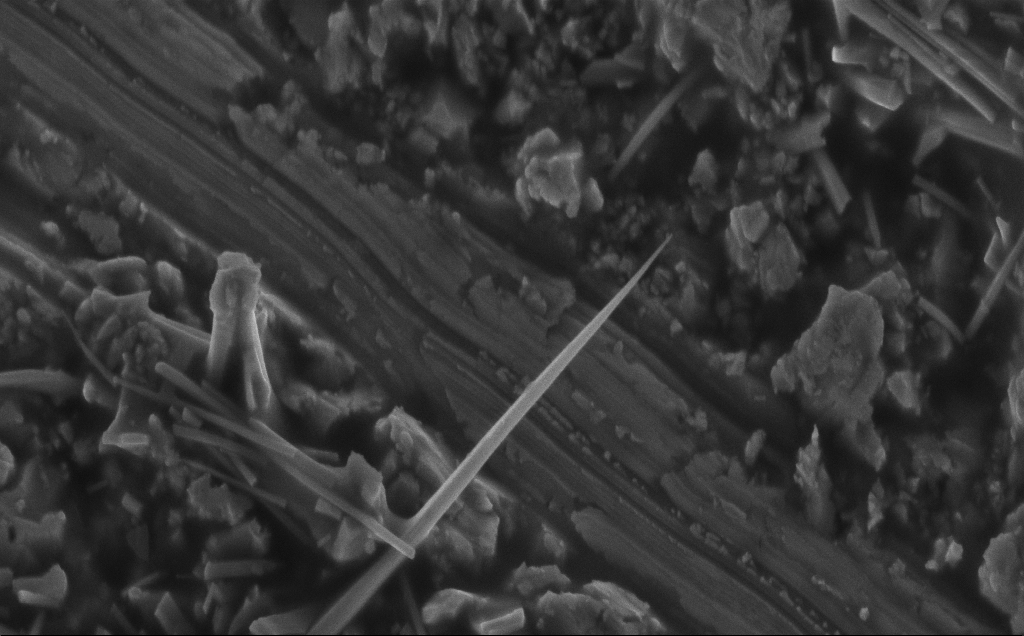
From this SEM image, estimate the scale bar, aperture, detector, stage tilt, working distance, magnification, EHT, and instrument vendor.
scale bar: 1000 nm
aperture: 30 µm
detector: InLens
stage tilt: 30°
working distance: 7 mm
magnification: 38.68 K X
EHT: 5 kV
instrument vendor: Zeiss